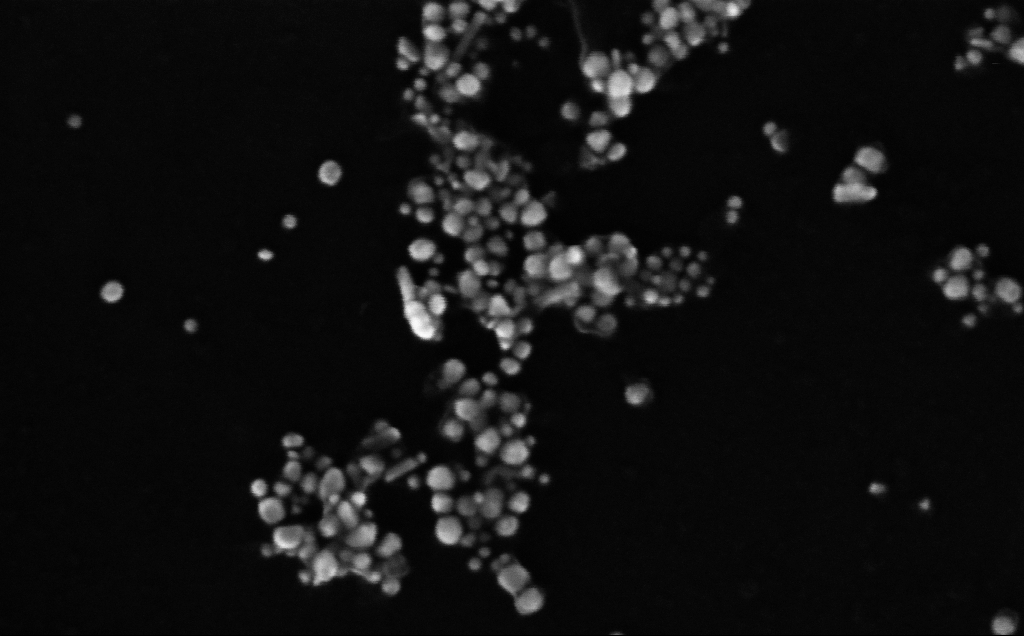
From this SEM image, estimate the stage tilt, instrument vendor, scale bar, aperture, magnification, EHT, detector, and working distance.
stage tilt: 0°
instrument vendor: Zeiss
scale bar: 100 nm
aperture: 30 µm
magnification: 365.74 K X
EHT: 10 kV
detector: InLens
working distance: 4 mm